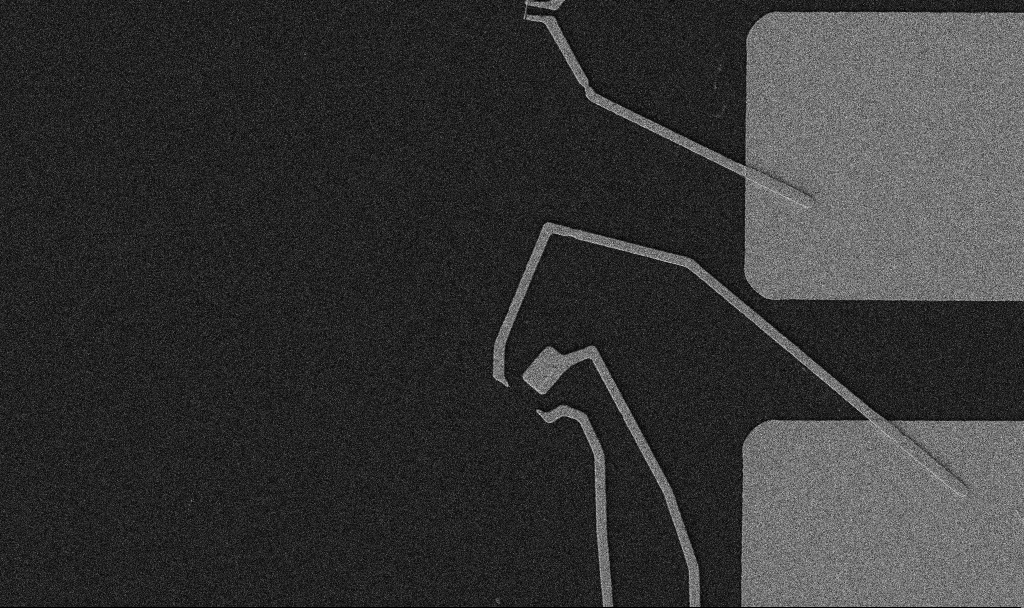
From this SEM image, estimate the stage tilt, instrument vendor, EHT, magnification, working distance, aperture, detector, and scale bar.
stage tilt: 0°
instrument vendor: Zeiss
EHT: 5 kV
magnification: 5 K X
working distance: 10.7 mm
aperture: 30 µm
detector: SE2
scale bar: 10000 nm